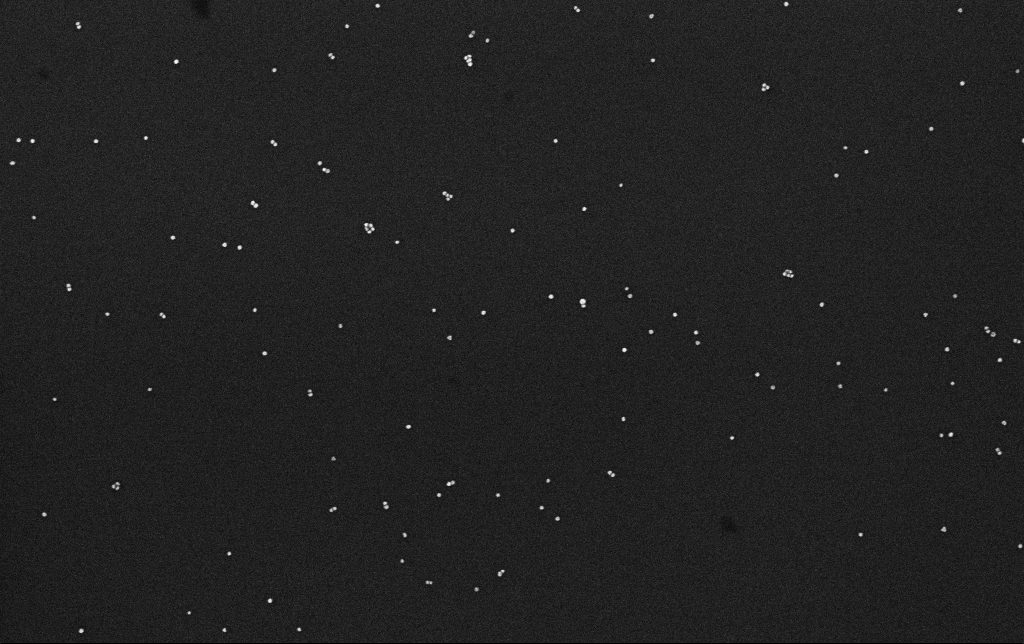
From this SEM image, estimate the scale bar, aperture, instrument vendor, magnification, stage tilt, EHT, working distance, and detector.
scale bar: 200 nm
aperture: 30 µm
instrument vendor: Zeiss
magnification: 100 K X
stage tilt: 0°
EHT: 10 kV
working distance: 3.1 mm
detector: InLens